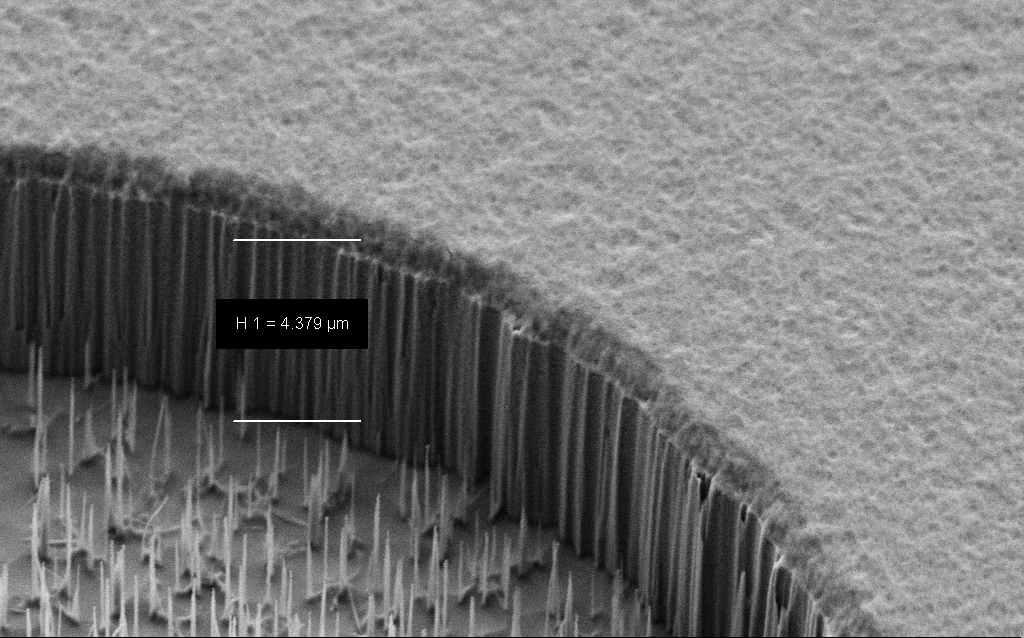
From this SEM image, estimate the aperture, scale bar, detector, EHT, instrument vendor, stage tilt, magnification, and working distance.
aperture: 30 µm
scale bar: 2000 nm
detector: SE2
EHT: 3 kV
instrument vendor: Zeiss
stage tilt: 45°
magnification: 15.18 K X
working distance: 7 mm